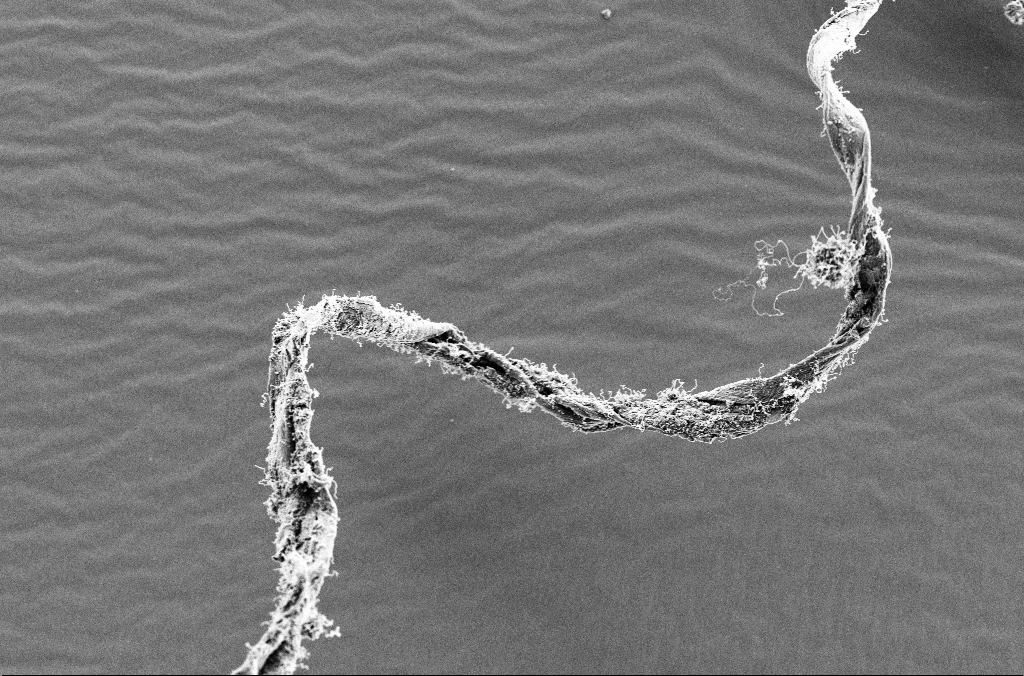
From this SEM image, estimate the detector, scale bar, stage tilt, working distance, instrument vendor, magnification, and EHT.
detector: SE2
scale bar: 10000 nm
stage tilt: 0°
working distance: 5 mm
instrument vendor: Zeiss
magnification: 2.5 K X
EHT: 3 kV